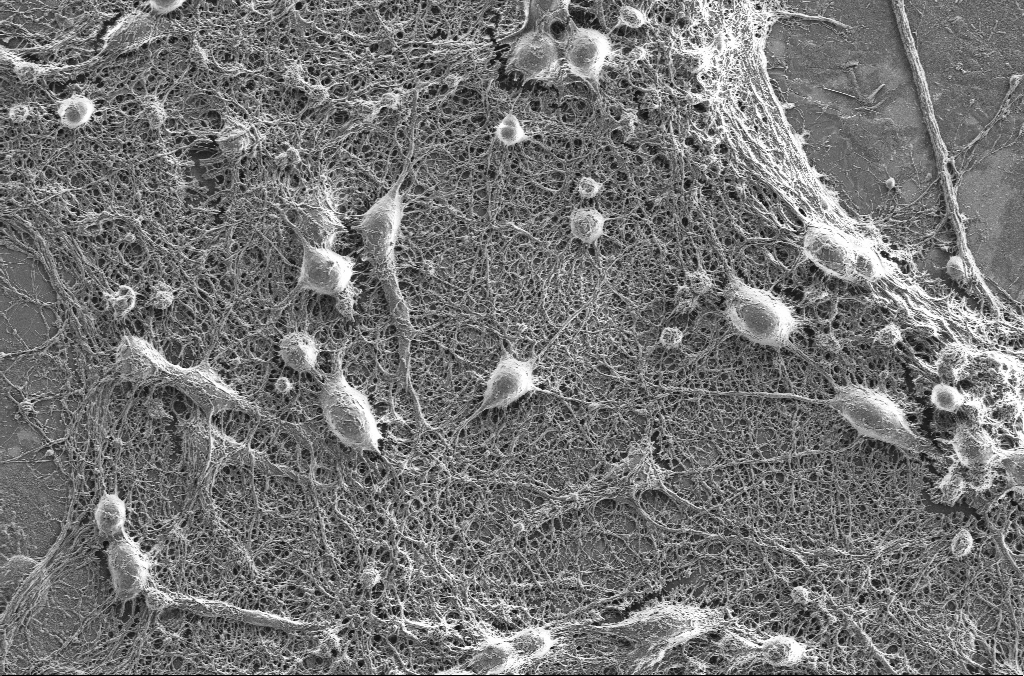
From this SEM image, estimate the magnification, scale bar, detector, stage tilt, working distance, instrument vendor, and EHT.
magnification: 2.5 K X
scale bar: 10000 nm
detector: SE2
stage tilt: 0°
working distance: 4.1 mm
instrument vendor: Zeiss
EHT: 2 kV